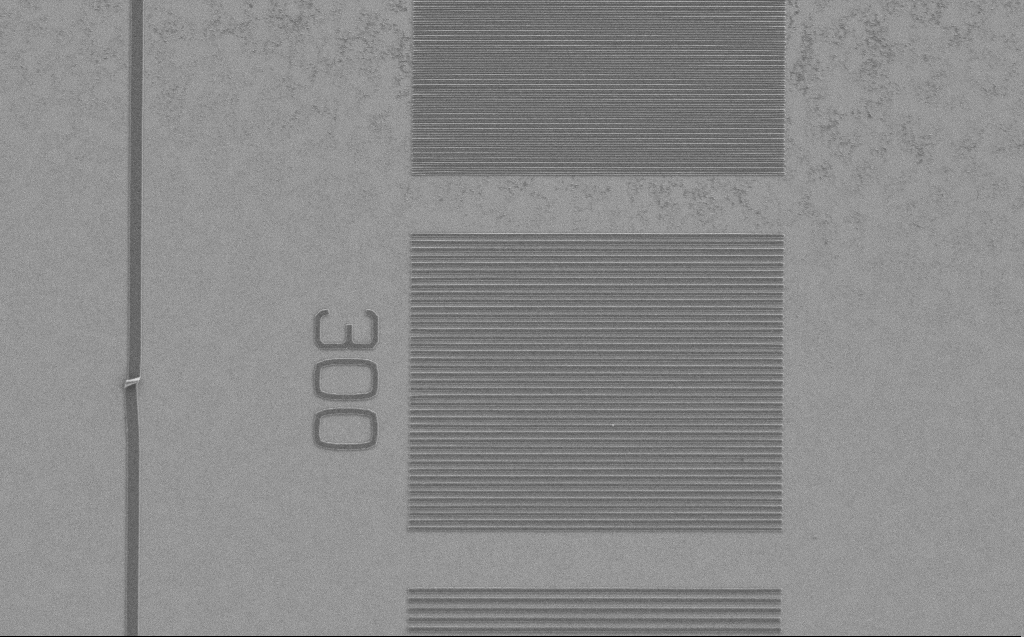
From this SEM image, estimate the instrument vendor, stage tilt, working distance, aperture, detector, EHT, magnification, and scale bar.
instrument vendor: Zeiss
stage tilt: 0°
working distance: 5 mm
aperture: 30 µm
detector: SE2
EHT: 1.2 kV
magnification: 4.54 K X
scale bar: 10000 nm